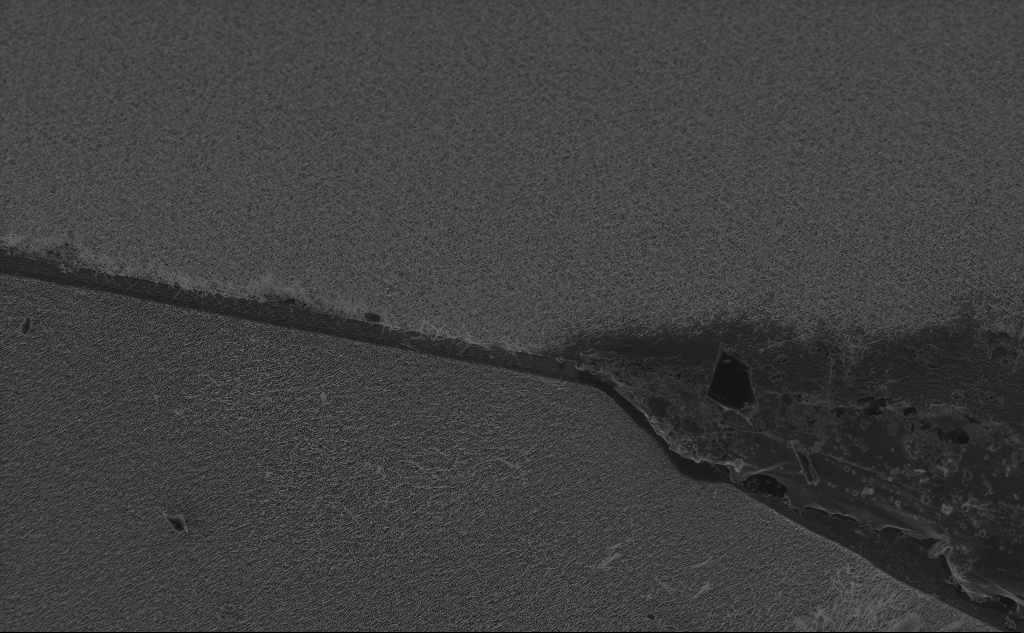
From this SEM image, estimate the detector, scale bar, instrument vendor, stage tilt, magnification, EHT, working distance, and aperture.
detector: InLens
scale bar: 20000 nm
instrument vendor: Zeiss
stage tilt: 45°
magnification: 1.56 K X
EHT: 10 kV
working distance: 5 mm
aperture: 30 µm